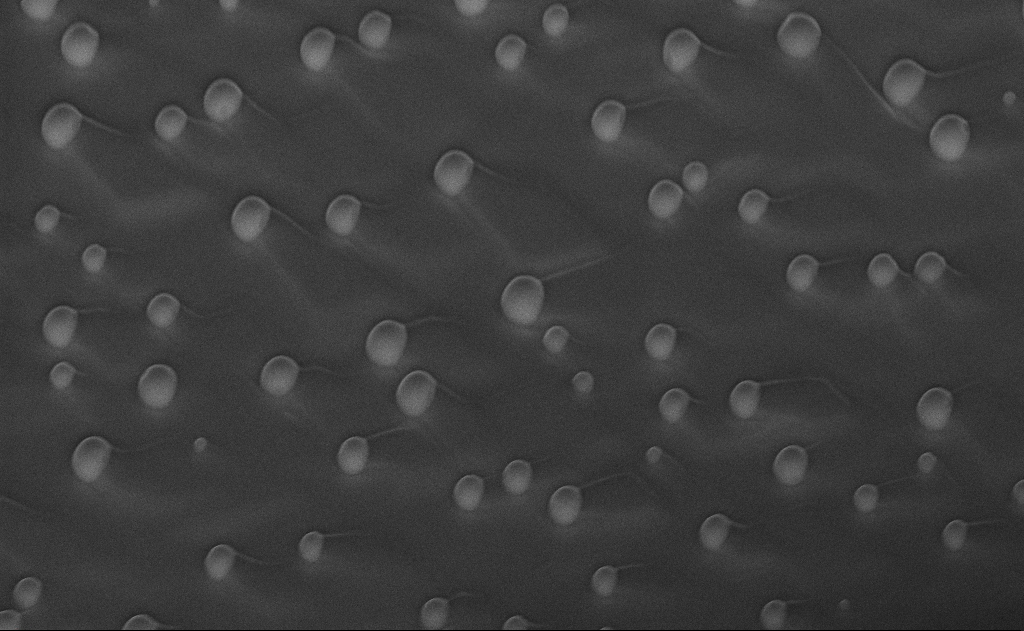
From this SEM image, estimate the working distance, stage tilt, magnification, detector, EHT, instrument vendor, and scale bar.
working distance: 7 mm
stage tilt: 48.9°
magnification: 13.93 K X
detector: InLens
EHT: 10 kV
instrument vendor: Zeiss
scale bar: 2000 nm